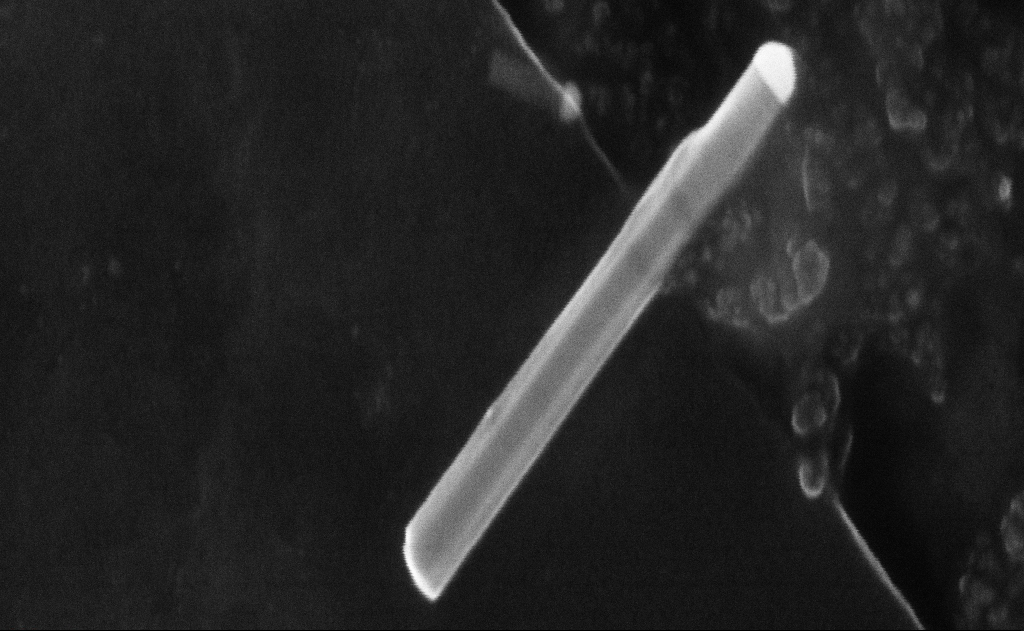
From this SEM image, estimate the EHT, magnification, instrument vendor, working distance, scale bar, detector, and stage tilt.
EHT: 20 kV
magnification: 263.3 K X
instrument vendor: Zeiss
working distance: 11 mm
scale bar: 200 nm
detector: InLens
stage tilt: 0°